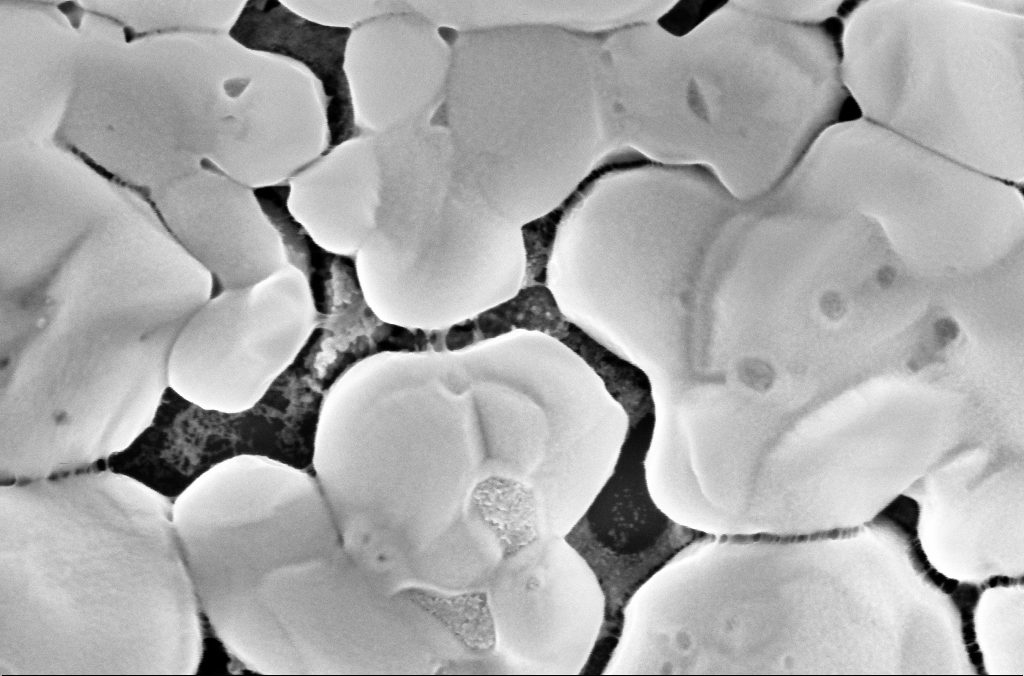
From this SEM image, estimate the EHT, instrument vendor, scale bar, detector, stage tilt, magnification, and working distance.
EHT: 5 kV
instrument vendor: Zeiss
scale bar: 200 nm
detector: InLens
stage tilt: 0°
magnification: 150 K X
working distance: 3.1 mm